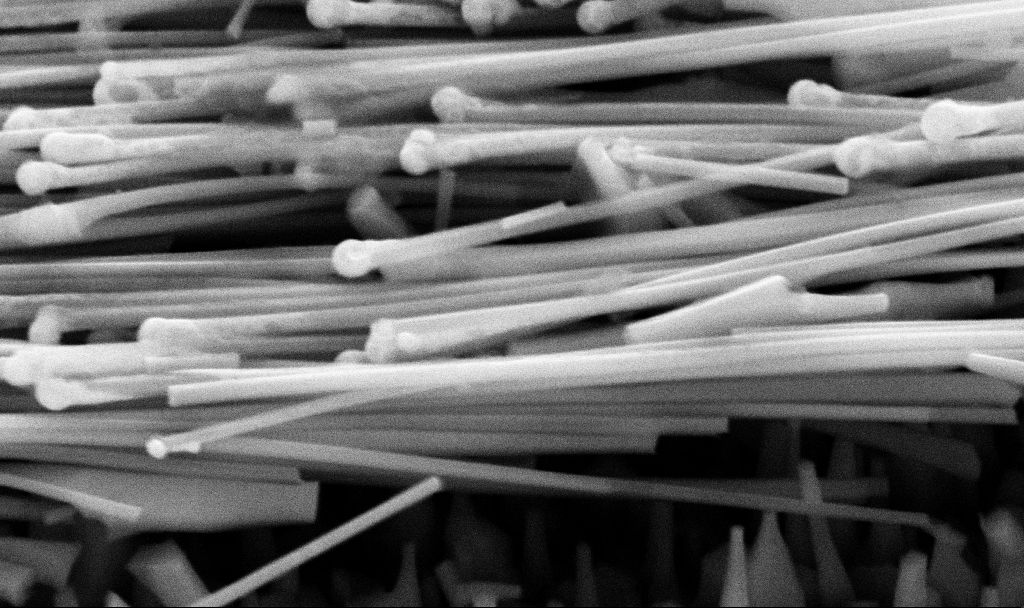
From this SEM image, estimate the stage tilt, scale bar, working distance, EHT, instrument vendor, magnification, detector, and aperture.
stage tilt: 45°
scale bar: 1000 nm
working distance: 11.2 mm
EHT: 10 kV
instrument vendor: Zeiss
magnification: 63.47 K X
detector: SE2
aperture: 30 µm